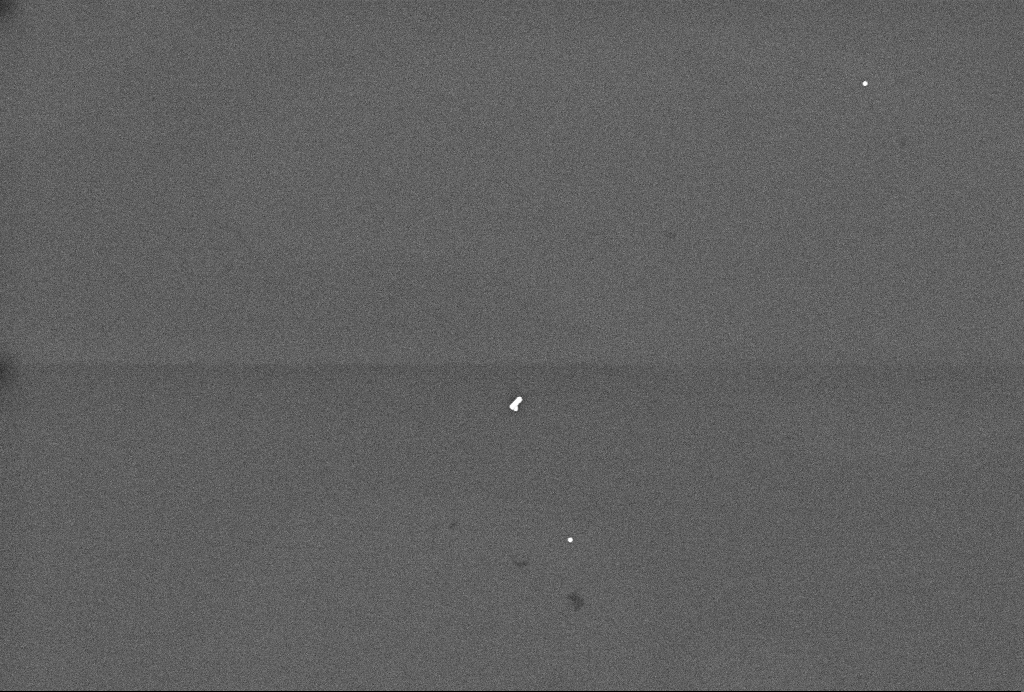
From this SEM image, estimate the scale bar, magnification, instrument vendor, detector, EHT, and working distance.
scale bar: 200 nm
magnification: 68.71 K X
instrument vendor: Zeiss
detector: InLens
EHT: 3 kV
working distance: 3.3 mm